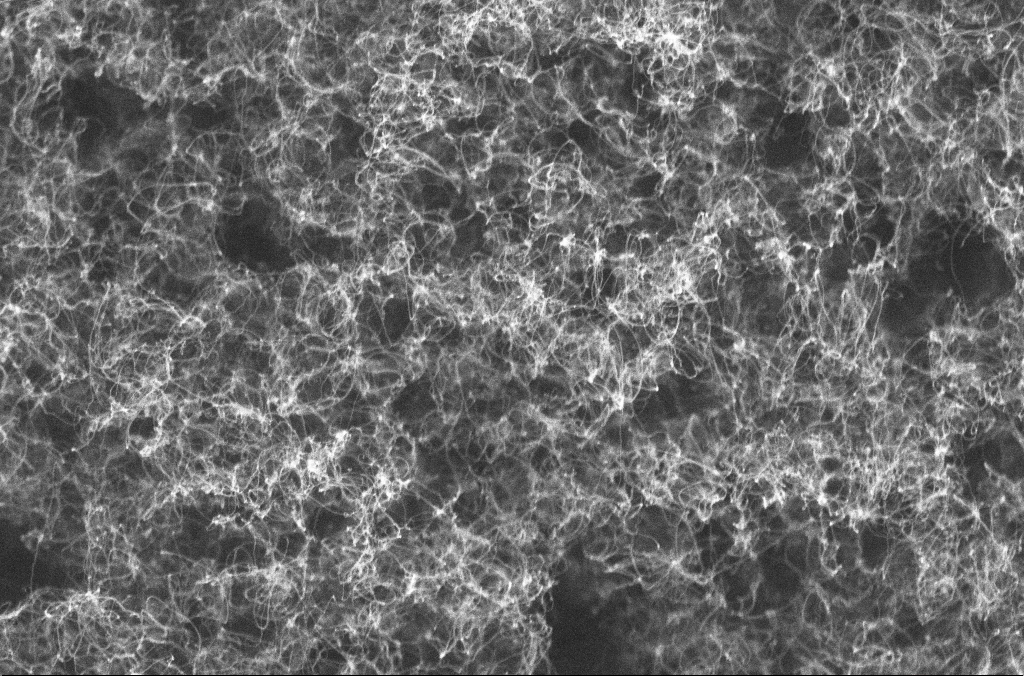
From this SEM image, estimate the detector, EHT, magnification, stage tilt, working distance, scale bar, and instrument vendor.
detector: InLens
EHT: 10 kV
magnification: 50 K X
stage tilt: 0°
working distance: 3.9 mm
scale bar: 1000 nm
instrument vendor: Zeiss